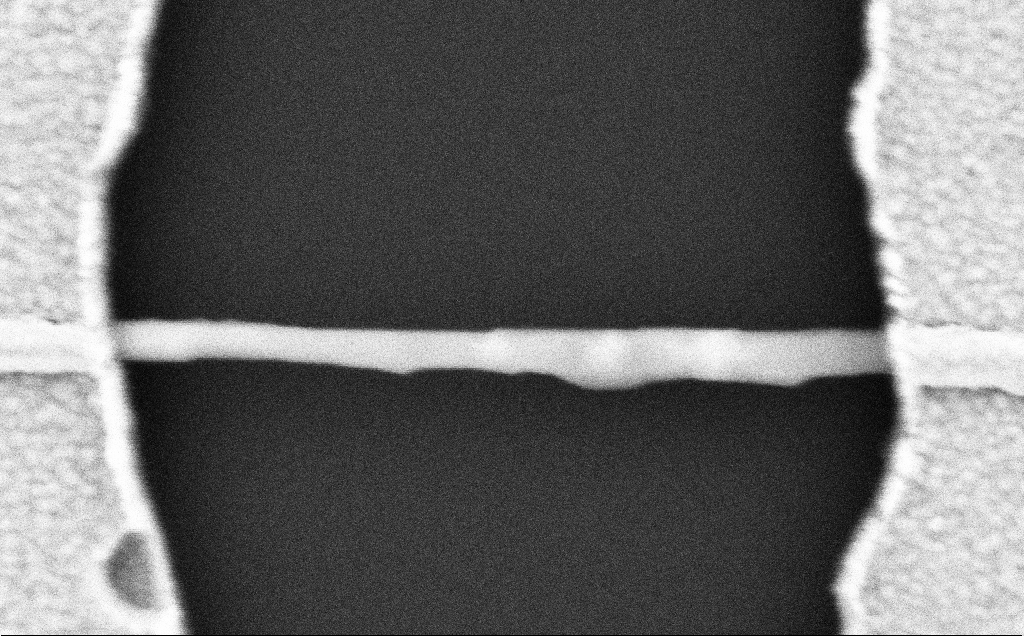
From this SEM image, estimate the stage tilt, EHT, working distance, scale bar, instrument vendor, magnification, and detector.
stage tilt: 0°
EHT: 5 kV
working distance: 10 mm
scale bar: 200 nm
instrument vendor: Zeiss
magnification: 155.87 K X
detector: SE2